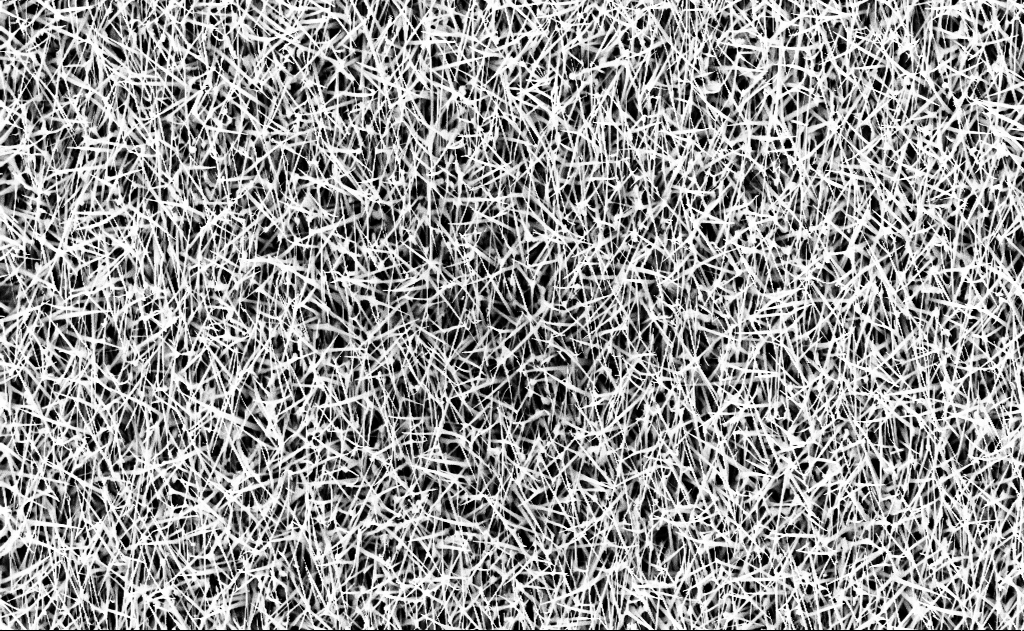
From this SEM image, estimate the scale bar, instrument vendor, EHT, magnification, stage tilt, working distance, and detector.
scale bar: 2000 nm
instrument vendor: Zeiss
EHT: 10 kV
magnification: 10 K X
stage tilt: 0°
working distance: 16 mm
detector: InLens